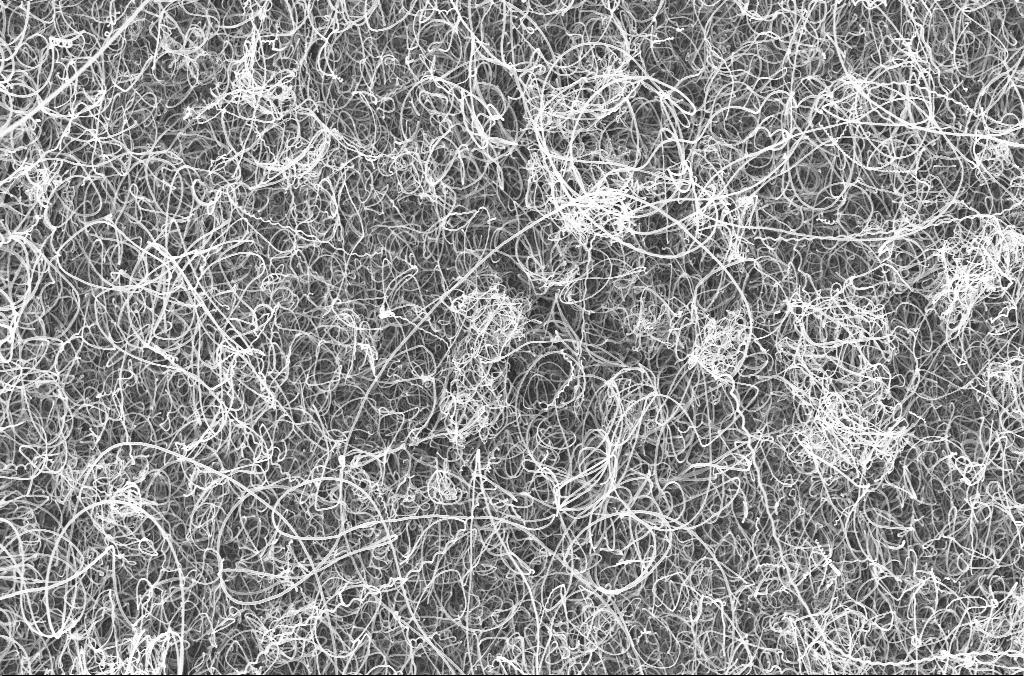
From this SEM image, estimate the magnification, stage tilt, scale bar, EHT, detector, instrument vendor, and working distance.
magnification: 15 K X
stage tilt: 0°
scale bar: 1000 nm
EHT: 20 kV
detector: InLens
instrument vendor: Zeiss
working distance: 4.2 mm